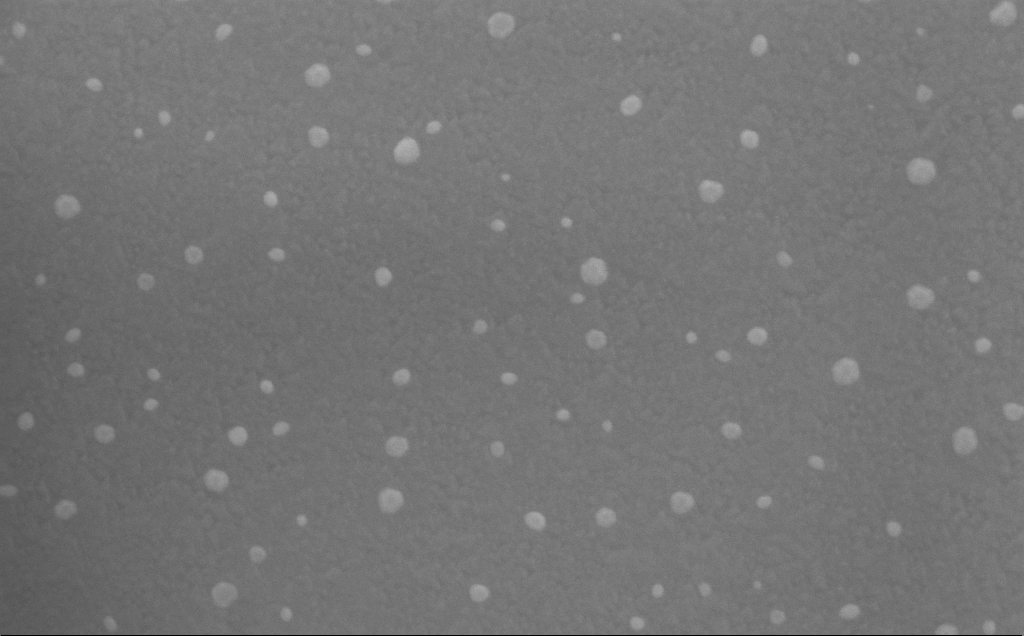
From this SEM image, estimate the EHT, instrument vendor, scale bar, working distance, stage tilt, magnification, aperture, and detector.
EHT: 10 kV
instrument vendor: Zeiss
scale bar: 200 nm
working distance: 5 mm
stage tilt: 15.5°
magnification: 172.67 K X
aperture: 30 µm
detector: InLens